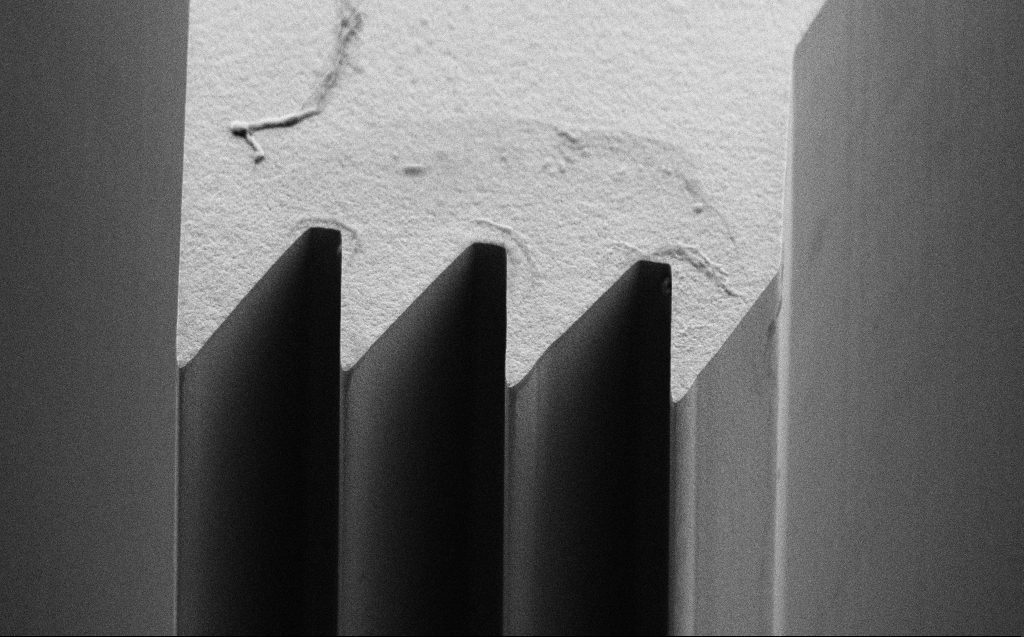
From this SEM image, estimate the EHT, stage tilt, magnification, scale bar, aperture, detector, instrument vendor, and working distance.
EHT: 5 kV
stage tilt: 45°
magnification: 5.42 K X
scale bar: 10000 nm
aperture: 30 µm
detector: SE2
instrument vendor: Zeiss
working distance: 5 mm